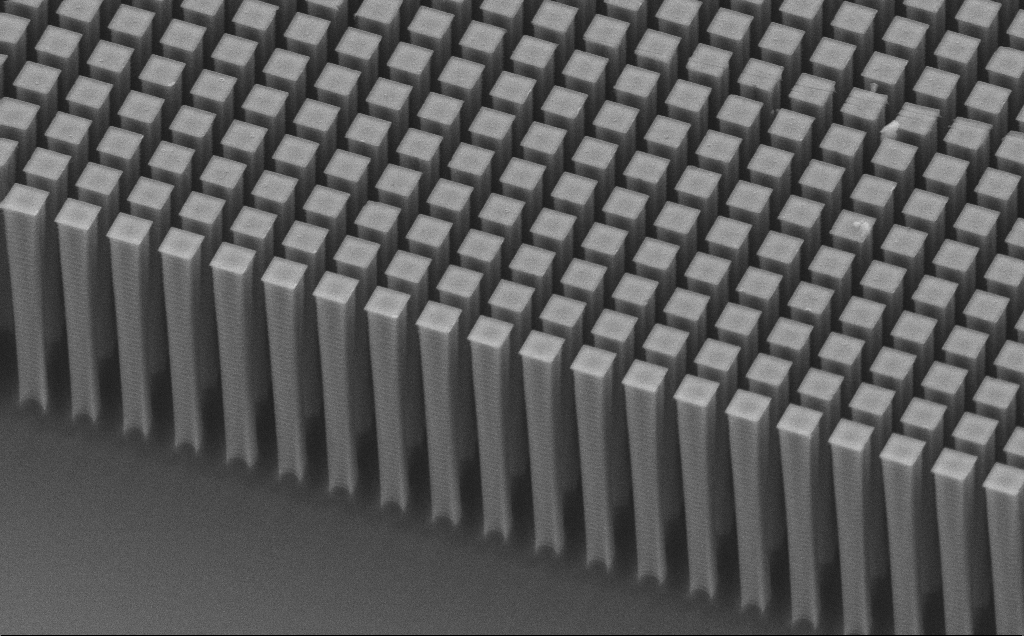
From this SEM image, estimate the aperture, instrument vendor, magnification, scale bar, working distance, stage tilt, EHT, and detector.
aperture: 30 µm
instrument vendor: Zeiss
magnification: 6.84 K X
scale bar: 10000 nm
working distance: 8 mm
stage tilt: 45°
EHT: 10 kV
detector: SE2